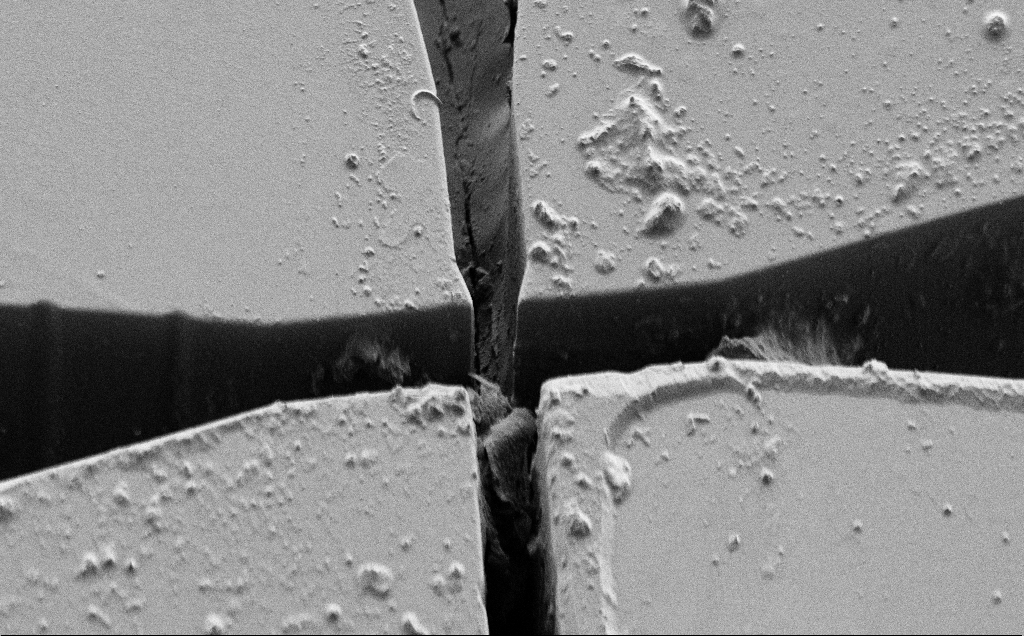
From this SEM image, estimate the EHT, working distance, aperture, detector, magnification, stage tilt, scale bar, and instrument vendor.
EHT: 1 kV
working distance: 6 mm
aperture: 30 µm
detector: SE2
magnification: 2.1 K X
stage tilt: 45°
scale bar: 20000 nm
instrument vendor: Zeiss